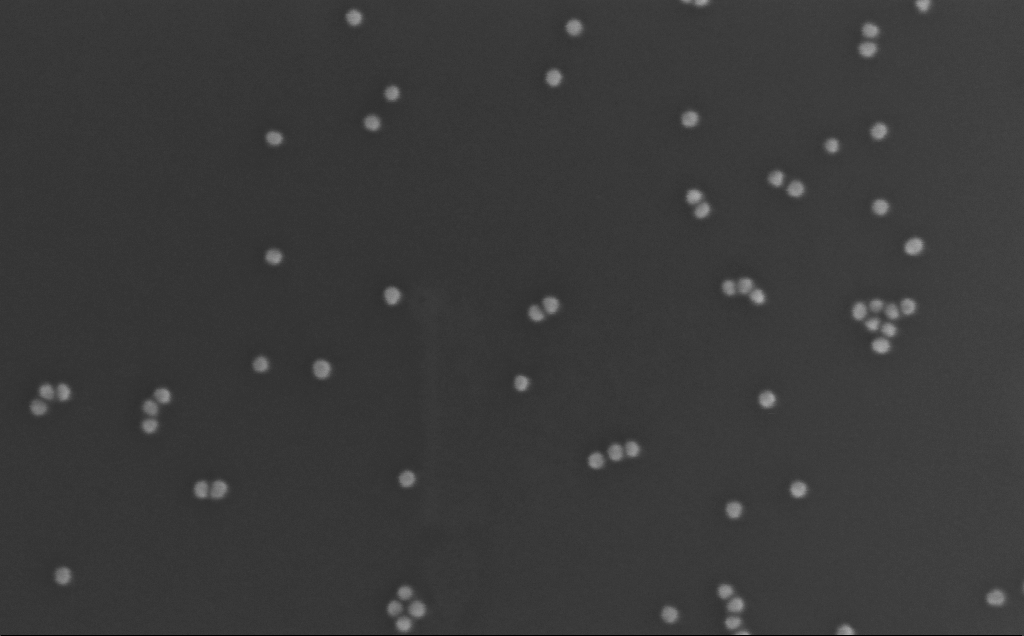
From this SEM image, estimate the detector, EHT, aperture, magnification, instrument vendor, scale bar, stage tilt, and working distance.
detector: InLens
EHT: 10 kV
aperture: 30 µm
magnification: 361.17 K X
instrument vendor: Zeiss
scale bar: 100 nm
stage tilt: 0°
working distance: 3 mm